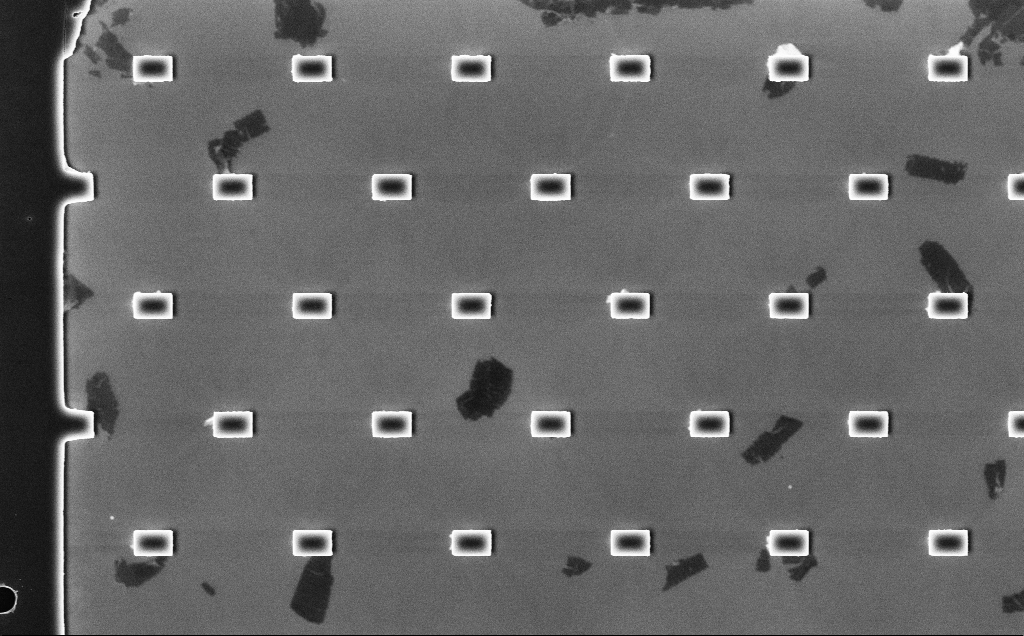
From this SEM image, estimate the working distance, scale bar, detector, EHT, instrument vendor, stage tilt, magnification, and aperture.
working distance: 3.2 mm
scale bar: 10000 nm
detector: InLens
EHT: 10 kV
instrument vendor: Zeiss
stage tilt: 0°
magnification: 4.86 K X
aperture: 30 µm